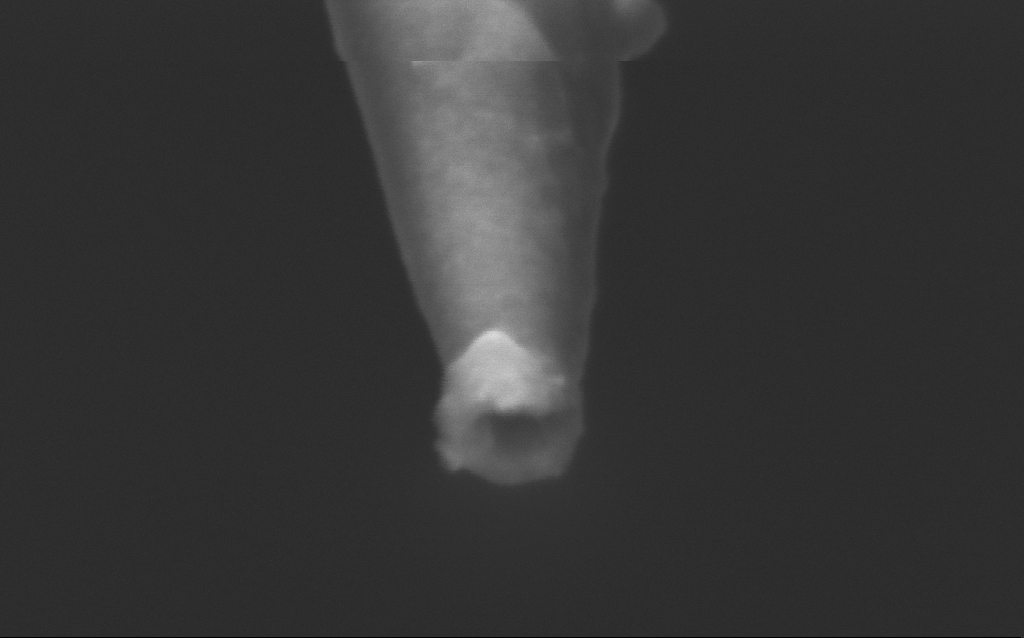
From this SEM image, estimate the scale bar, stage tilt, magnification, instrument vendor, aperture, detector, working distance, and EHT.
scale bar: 100 nm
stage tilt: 45°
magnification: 500 K X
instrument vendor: Zeiss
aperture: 30 µm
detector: InLens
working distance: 5 mm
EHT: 2.5 kV